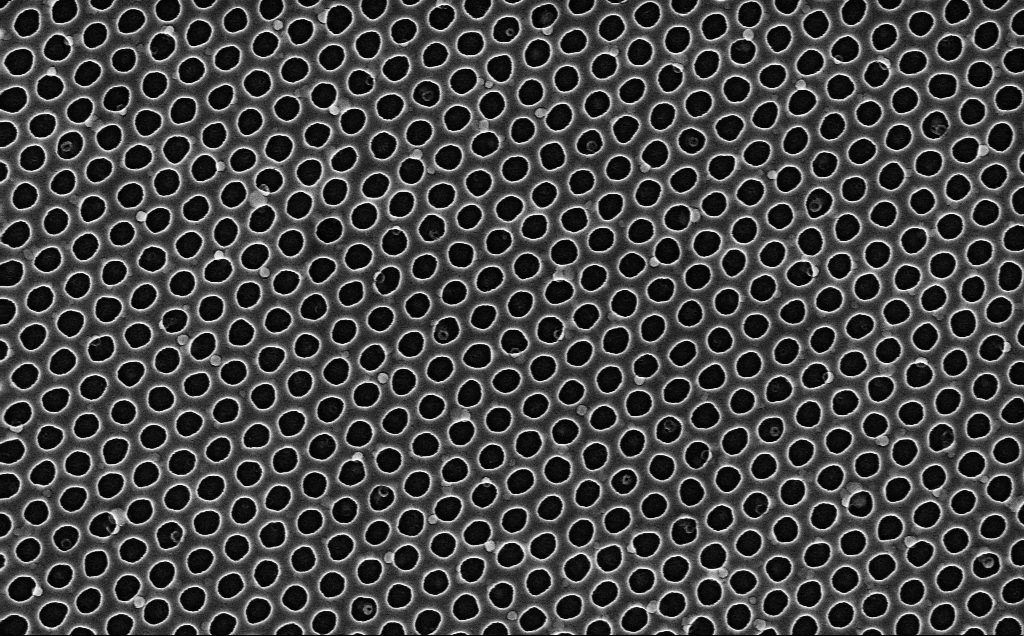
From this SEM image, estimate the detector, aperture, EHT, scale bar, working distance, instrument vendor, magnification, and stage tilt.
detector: InLens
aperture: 30 µm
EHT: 3 kV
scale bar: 1000 nm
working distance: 2.4 mm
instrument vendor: Zeiss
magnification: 40 K X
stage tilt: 0°